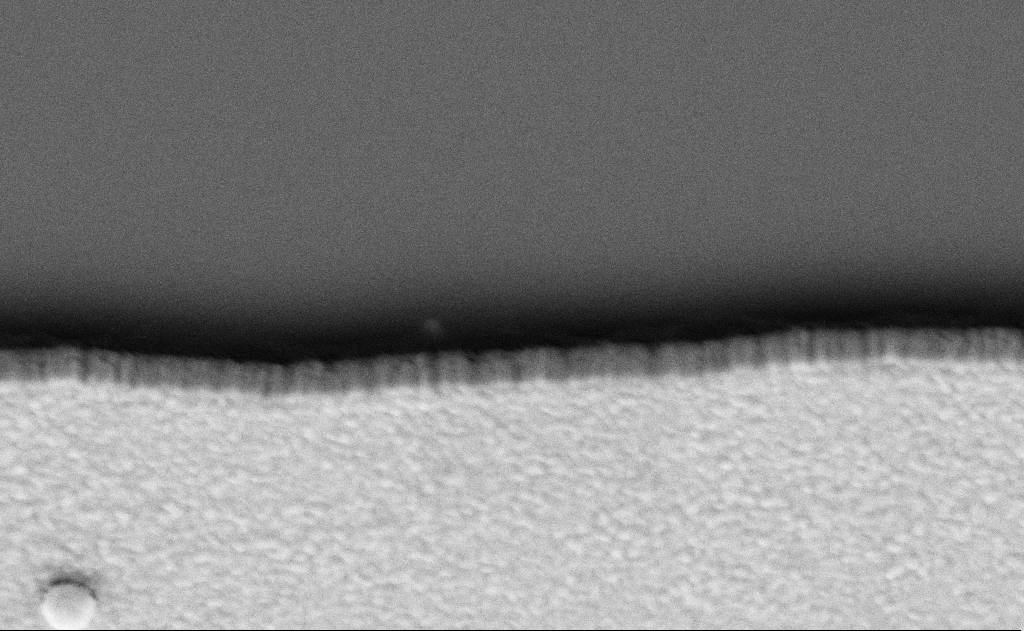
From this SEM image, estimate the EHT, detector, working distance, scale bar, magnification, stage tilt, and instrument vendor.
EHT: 5 kV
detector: SE2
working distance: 5 mm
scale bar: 200 nm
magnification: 117.32 K X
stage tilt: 40°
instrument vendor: Zeiss